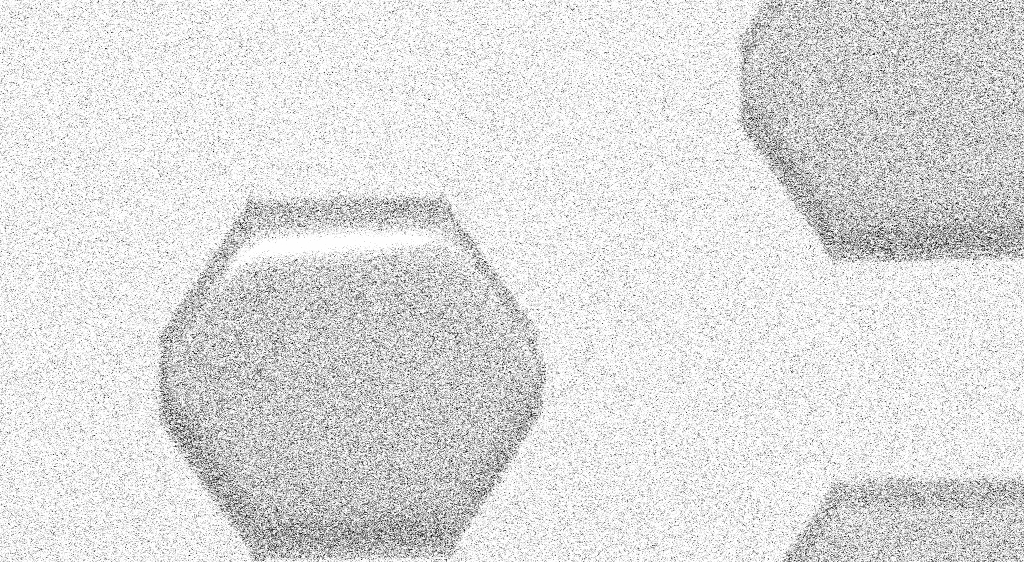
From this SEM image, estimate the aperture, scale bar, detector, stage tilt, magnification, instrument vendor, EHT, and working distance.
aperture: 30 µm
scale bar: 20000 nm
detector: SE2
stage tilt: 30°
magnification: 1.23 K X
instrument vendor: Zeiss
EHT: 1.5 kV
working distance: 6 mm